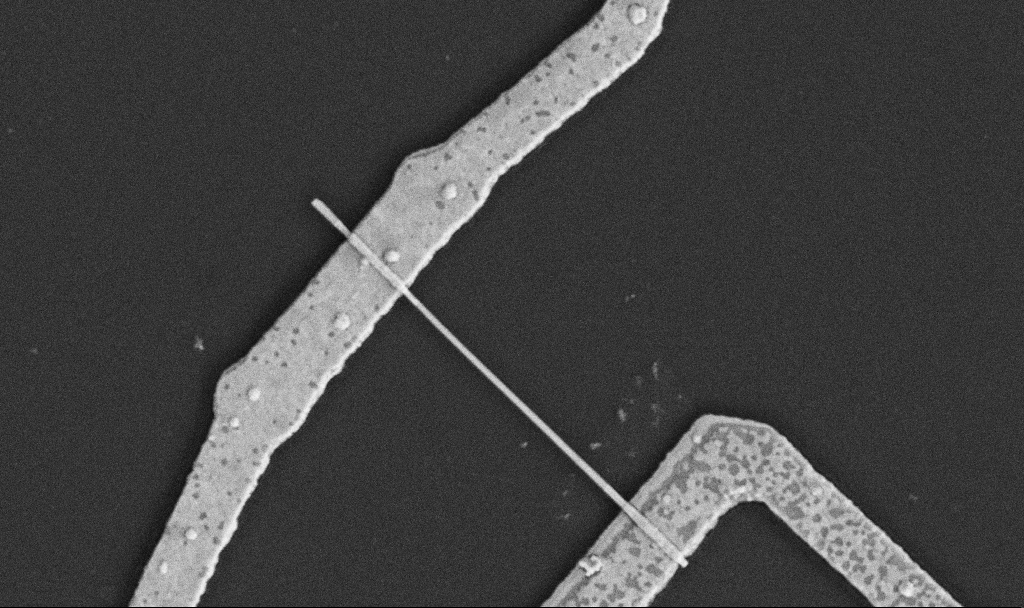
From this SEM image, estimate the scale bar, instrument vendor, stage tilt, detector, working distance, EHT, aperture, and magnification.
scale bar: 1000 nm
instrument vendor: Zeiss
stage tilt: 0°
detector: SE2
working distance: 10.6 mm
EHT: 5 kV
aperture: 30 µm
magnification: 30 K X